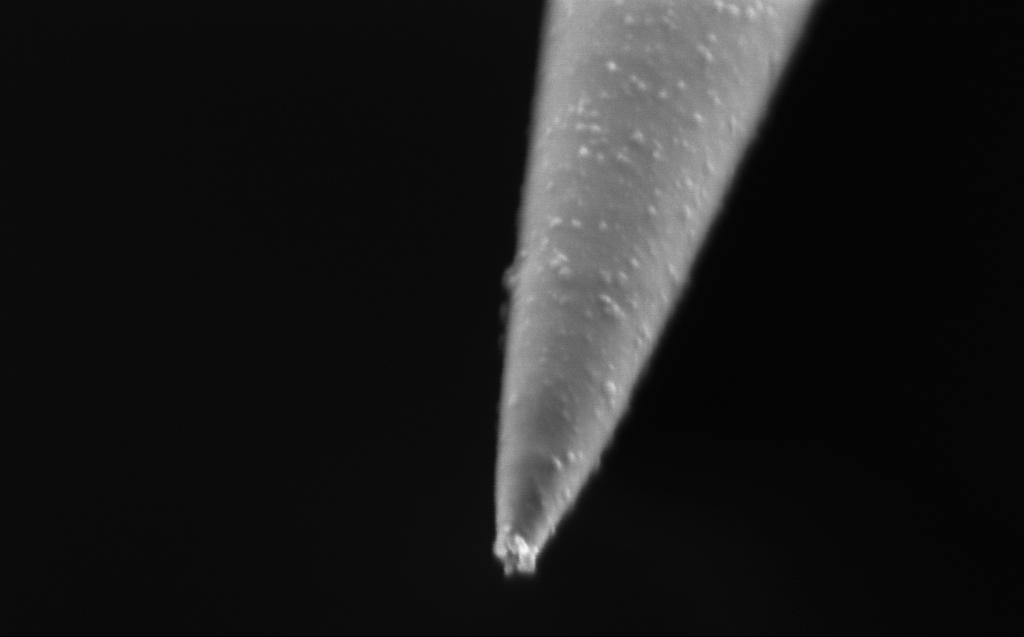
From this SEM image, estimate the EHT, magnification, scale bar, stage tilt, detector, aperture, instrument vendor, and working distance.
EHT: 0.8 kV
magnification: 137.05 K X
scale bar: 200 nm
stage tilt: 45°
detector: InLens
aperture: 30 µm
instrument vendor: Zeiss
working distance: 3 mm